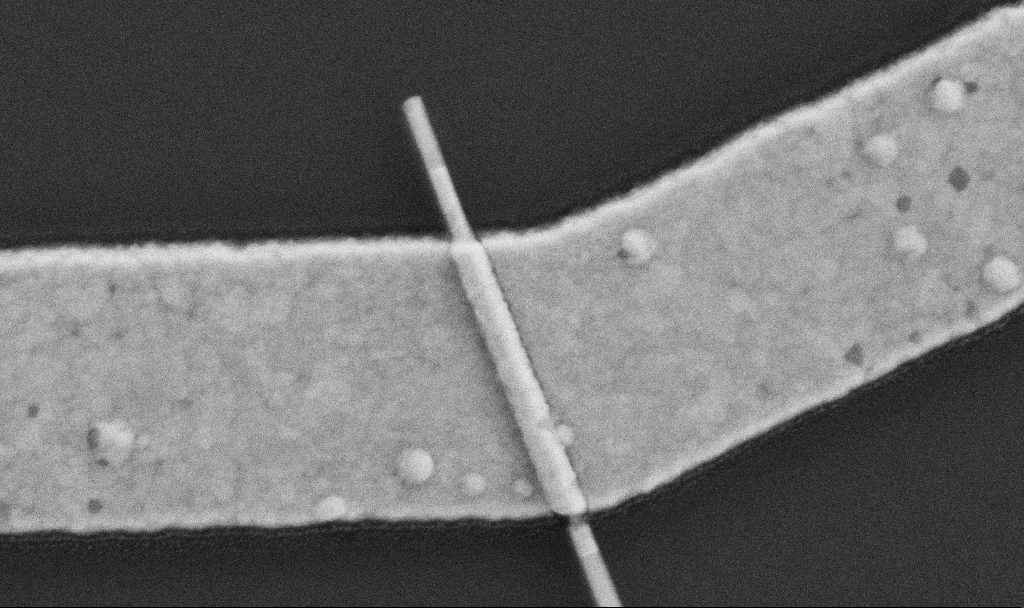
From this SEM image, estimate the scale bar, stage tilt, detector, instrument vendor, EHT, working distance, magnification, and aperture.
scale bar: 200 nm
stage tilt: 0°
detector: SE2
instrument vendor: Zeiss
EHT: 5 kV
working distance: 7.6 mm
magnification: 100 K X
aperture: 30 µm